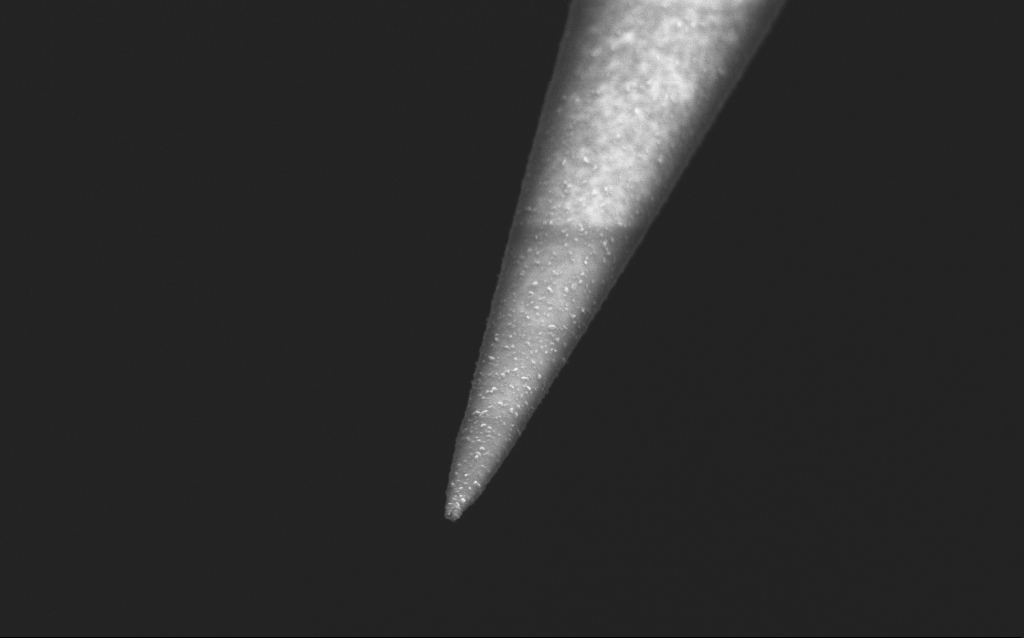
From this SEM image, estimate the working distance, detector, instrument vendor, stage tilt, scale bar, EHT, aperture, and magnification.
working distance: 5 mm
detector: InLens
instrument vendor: Zeiss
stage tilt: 45°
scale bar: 1000 nm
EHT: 2.5 kV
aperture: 30 µm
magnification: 50 K X